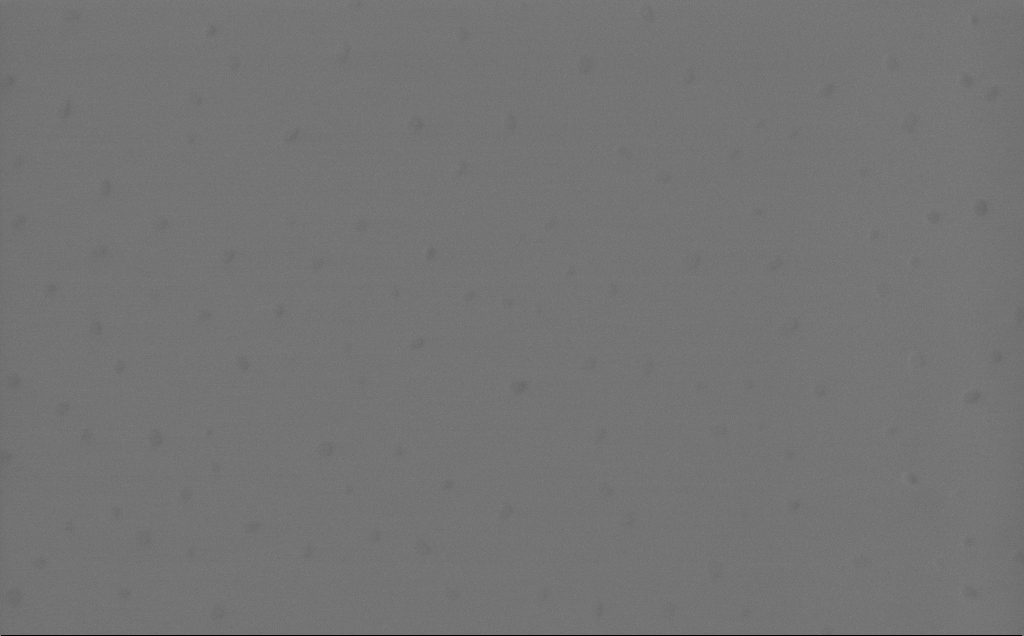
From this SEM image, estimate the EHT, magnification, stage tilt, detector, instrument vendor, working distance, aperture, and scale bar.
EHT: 1 kV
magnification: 75.64 K X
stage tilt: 0°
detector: InLens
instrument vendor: Zeiss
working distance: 3 mm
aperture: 30 µm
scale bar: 200 nm